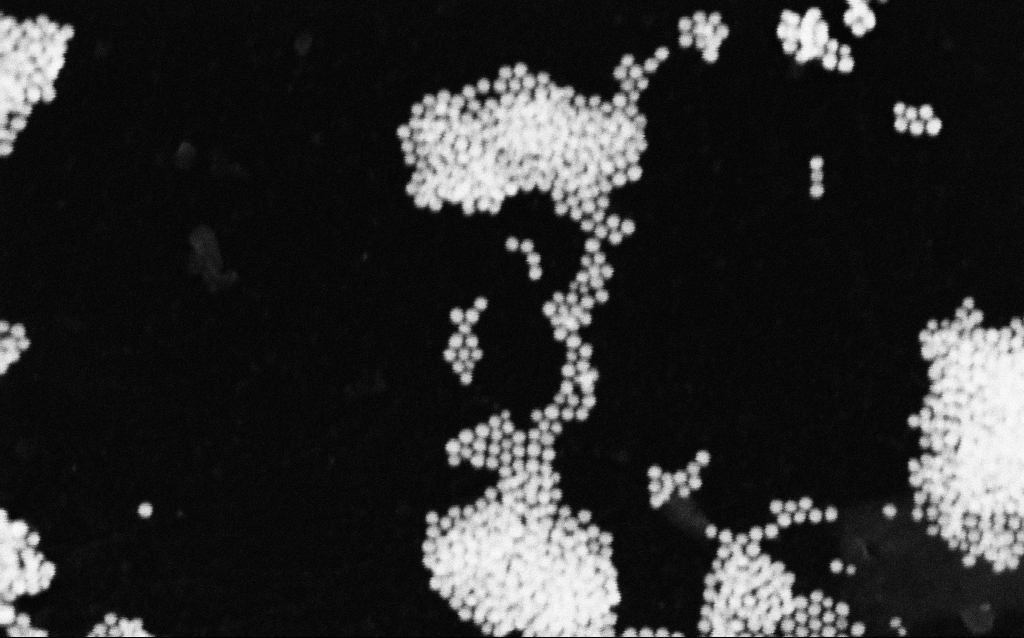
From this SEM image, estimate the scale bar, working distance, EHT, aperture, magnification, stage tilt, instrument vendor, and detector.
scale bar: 100 nm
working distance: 12.9 mm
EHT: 30 kV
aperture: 30 µm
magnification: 252.89 K X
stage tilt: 0°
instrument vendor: Zeiss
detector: SE2